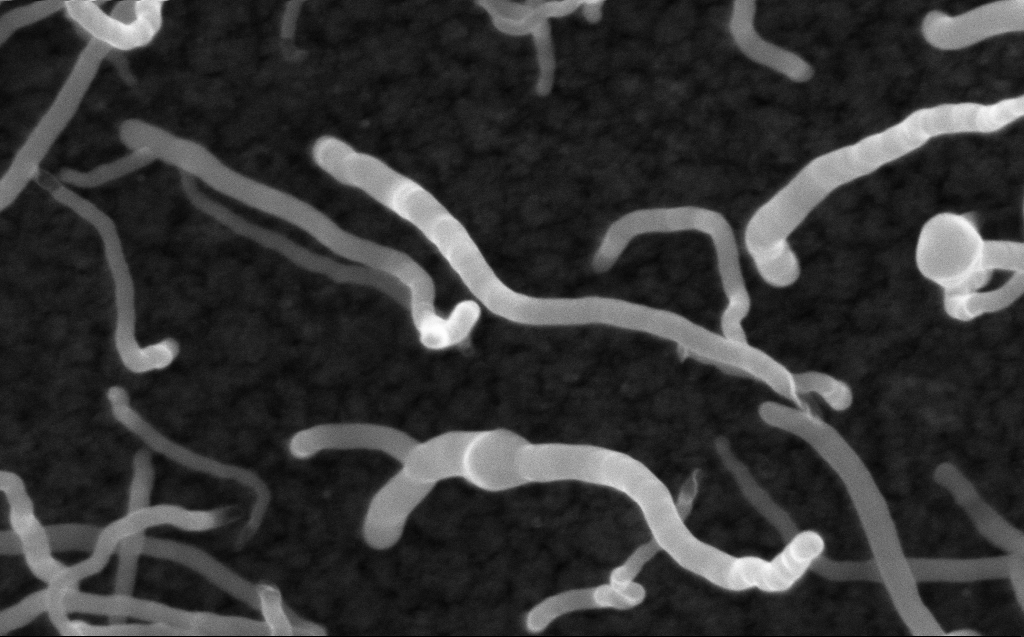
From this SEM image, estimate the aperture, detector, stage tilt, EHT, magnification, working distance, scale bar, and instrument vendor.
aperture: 30 µm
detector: InLens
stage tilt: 0°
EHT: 10 kV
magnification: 200 K X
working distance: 3 mm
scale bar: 200 nm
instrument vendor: Zeiss